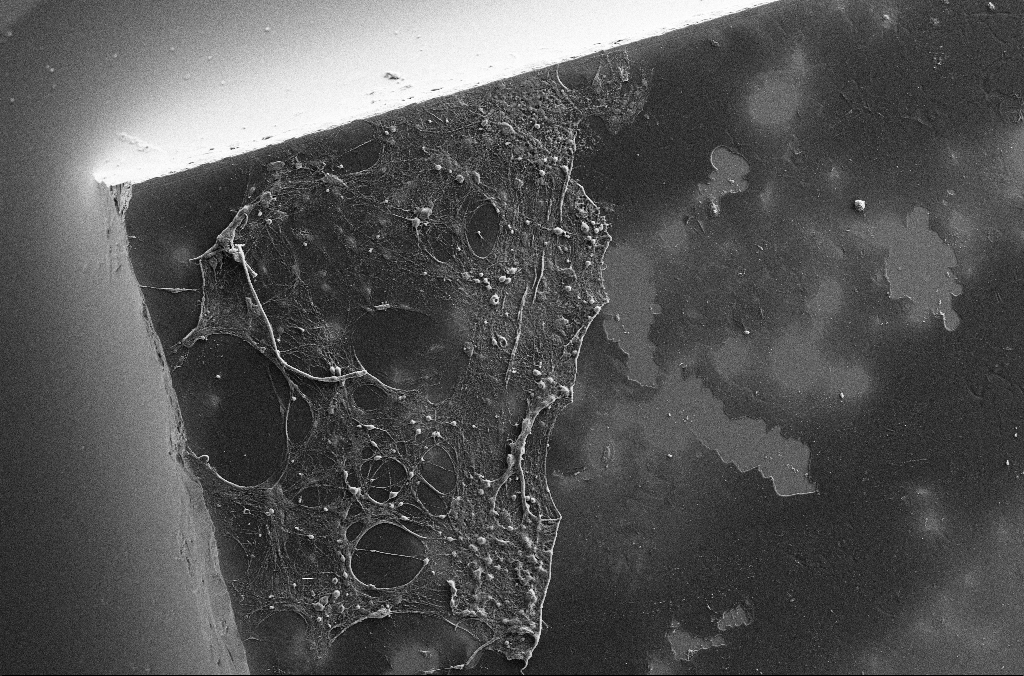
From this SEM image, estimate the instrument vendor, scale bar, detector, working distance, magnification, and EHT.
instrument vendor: Zeiss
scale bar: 100000 nm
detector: SE2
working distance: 3.1 mm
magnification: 0.2 K X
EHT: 15 kV